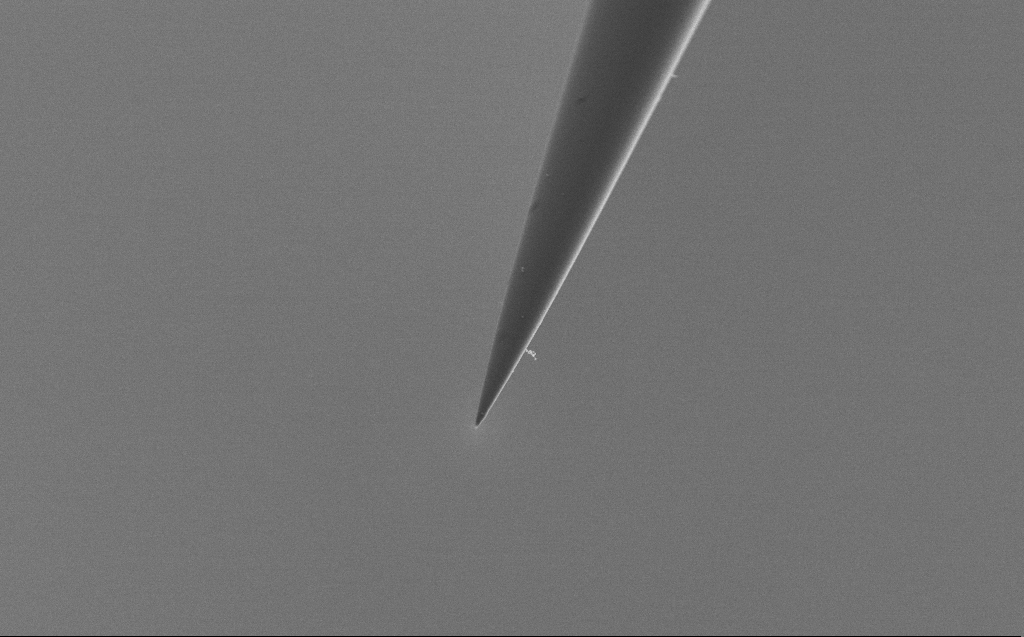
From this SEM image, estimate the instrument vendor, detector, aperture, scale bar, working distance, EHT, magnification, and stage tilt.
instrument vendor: Zeiss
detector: SE2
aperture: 30 µm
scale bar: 2000 nm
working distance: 4 mm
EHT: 2 kV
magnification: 10 K X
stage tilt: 45°